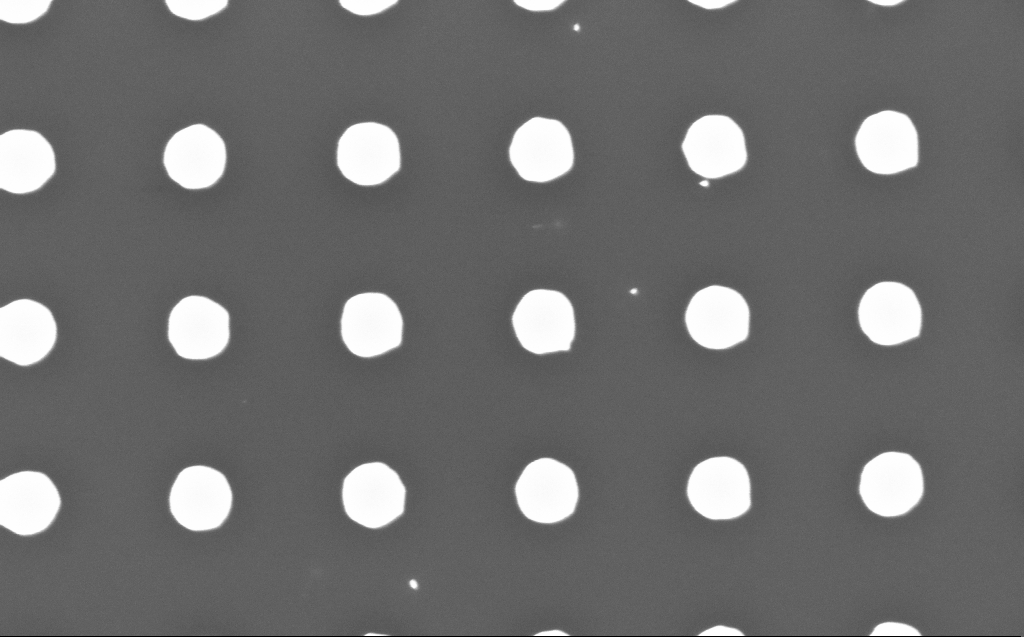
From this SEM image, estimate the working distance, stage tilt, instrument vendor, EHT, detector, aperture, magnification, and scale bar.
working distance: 5 mm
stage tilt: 0°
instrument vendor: Zeiss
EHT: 5 kV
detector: InLens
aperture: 30 µm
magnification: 60.63 K X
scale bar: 1000 nm